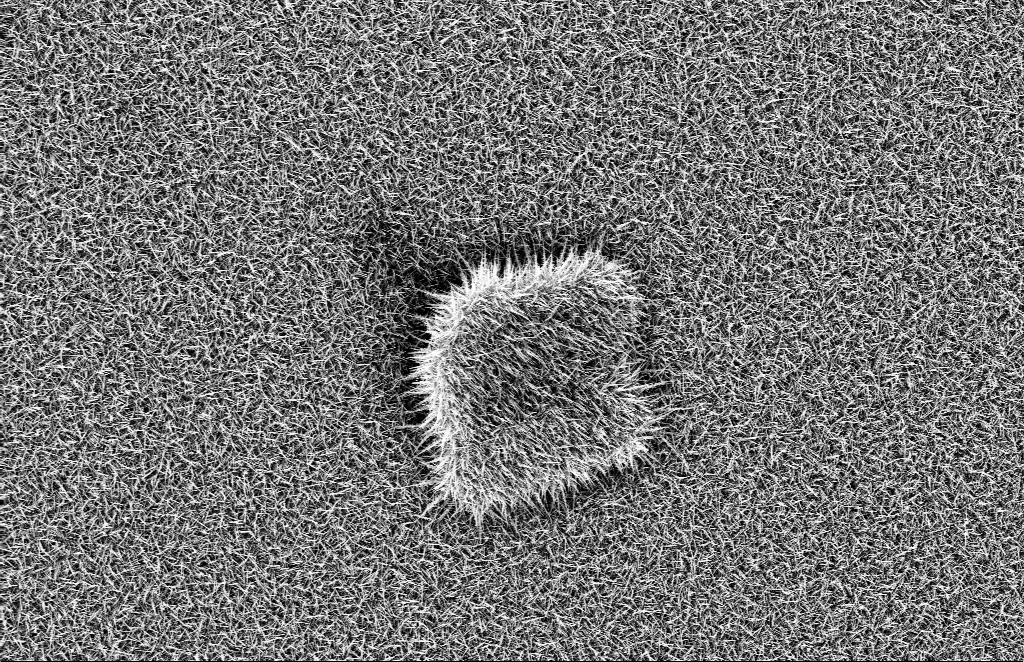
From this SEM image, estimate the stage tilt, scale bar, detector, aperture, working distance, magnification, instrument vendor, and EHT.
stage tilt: -1.1°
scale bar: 2000 nm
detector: InLens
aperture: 30 µm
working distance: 10 mm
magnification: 5 K X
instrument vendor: Zeiss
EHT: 10 kV